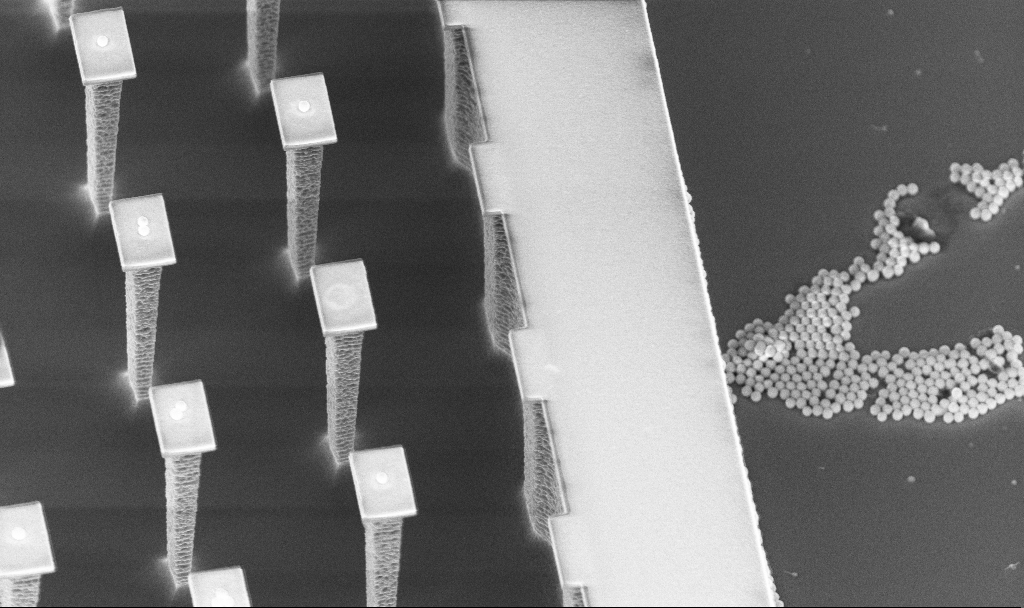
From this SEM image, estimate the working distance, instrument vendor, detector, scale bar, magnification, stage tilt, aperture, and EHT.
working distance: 4.5 mm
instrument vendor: Zeiss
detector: InLens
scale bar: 10000 nm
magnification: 6.74 K X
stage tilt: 30°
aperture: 30 µm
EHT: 5 kV